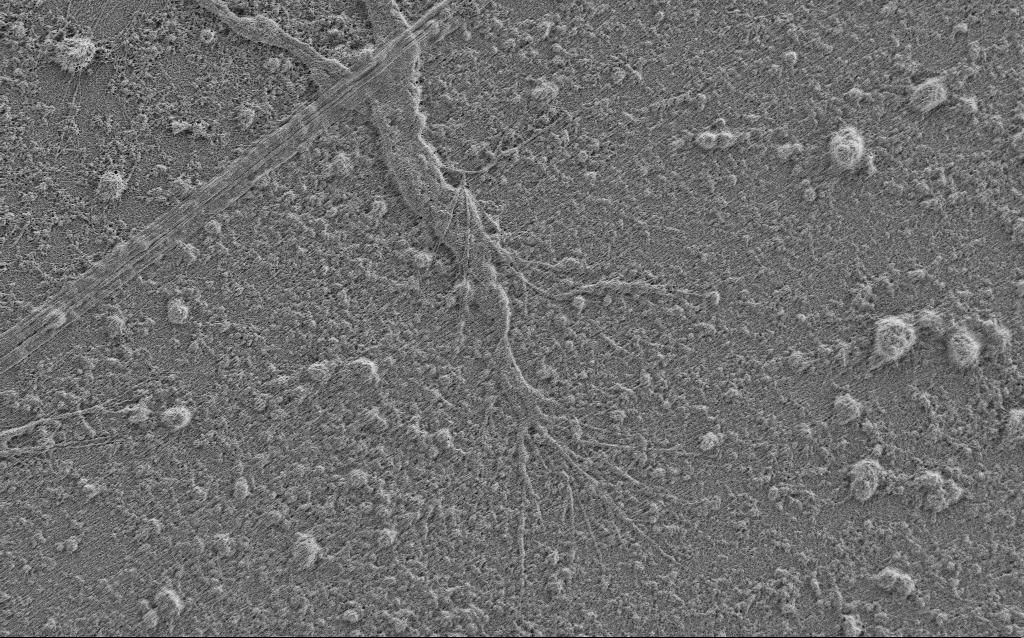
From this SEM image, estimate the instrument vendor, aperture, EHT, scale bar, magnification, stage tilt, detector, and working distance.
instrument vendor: Zeiss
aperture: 30 µm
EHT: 0.9 kV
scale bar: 10000 nm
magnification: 5 K X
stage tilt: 0°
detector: SE2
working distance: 4 mm